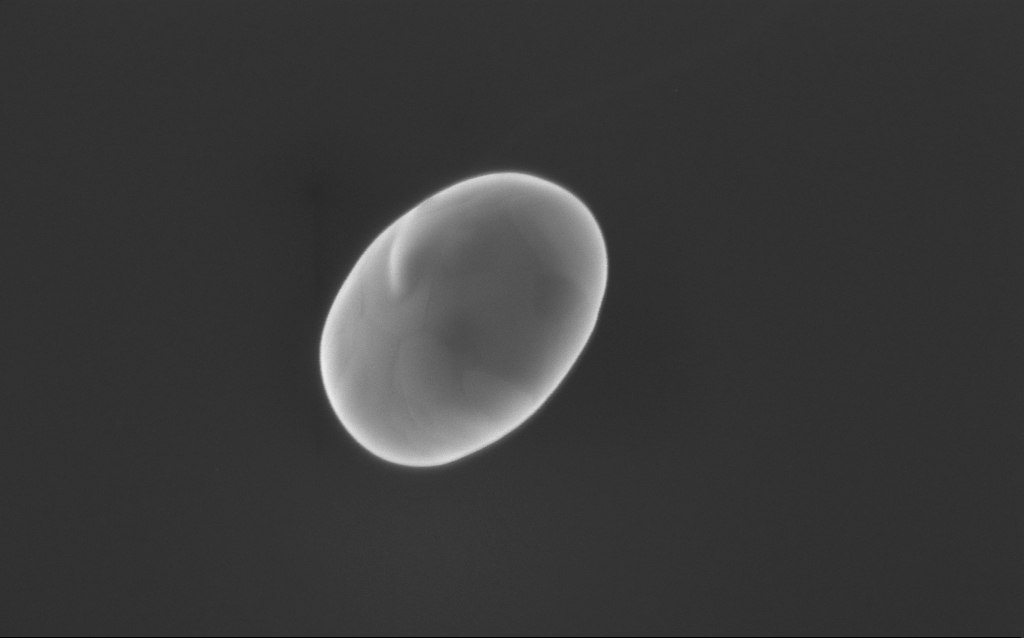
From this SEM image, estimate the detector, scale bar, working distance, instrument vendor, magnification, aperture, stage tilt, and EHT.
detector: InLens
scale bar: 200 nm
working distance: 6 mm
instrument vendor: Zeiss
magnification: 103.69 K X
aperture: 30 µm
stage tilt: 22°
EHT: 6 kV